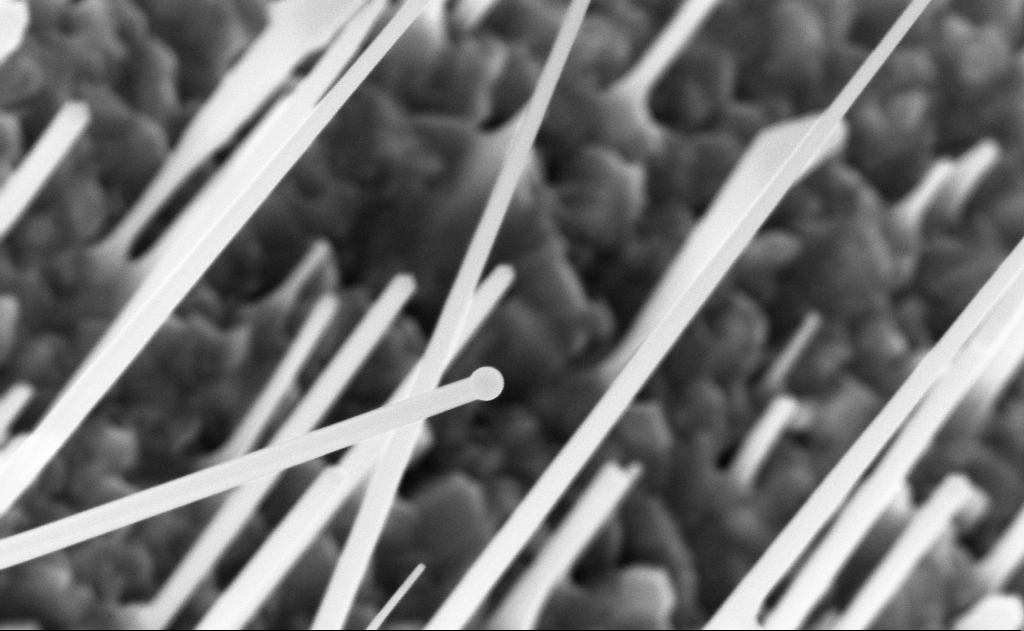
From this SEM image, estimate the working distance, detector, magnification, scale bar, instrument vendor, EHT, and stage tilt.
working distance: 10 mm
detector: InLens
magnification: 80 K X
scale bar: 200 nm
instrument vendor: Zeiss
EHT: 10 kV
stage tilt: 0°